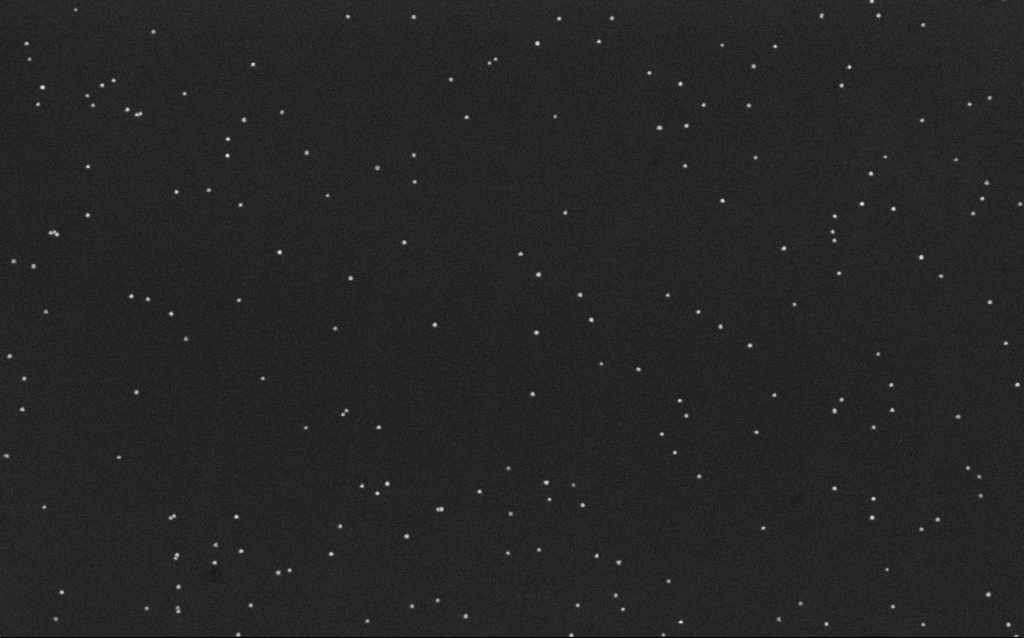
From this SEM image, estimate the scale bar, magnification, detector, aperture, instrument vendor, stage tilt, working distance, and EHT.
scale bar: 200 nm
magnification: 100 K X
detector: InLens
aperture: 30 µm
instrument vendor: Zeiss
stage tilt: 0°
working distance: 6.5 mm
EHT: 10 kV